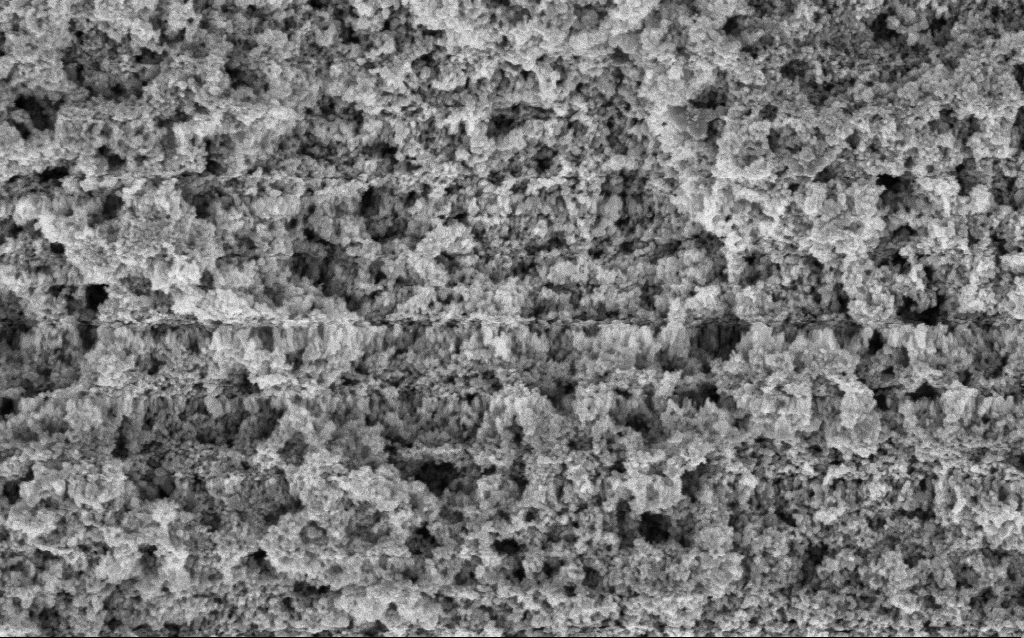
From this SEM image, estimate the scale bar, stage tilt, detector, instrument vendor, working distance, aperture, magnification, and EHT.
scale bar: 1000 nm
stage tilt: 0°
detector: InLens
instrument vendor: Zeiss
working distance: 10 mm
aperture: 30 µm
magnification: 65.04 K X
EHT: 3 kV